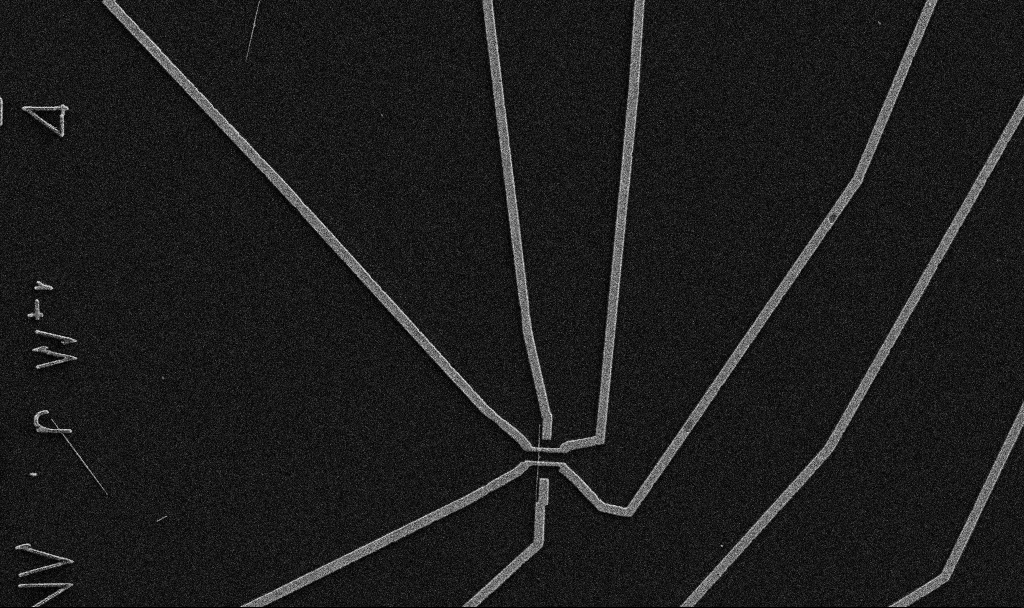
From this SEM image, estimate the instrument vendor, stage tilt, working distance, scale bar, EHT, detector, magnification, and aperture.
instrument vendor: Zeiss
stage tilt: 0°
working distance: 10.7 mm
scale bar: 10000 nm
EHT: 5 kV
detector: SE2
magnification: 5 K X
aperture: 30 µm